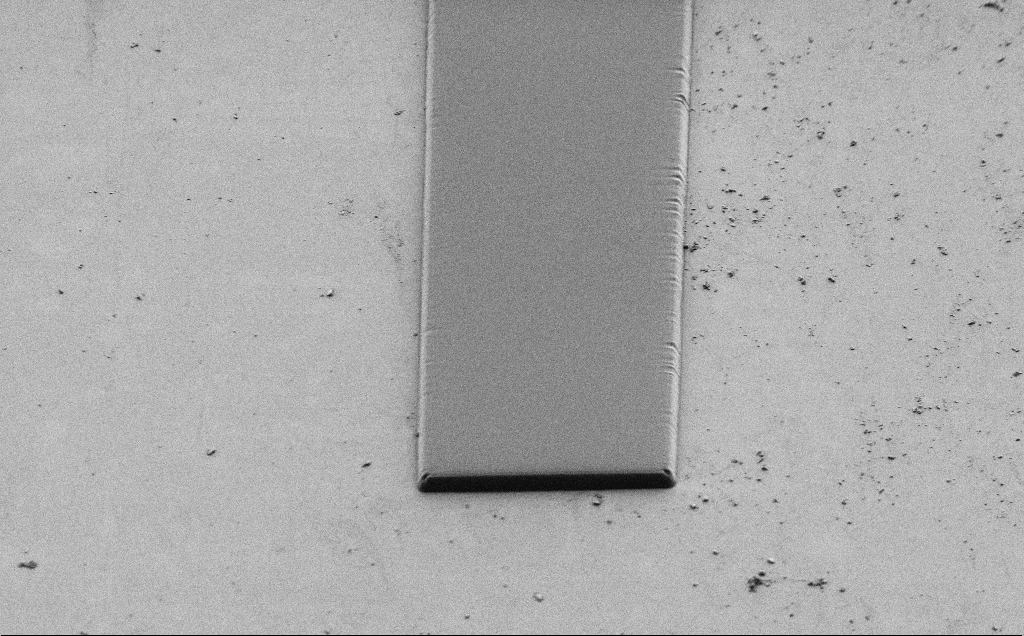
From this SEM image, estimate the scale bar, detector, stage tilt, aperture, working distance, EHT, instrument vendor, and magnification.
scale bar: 20000 nm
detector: SE2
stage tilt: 45°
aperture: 30 µm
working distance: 7 mm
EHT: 1 kV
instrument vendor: Zeiss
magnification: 1.09 K X